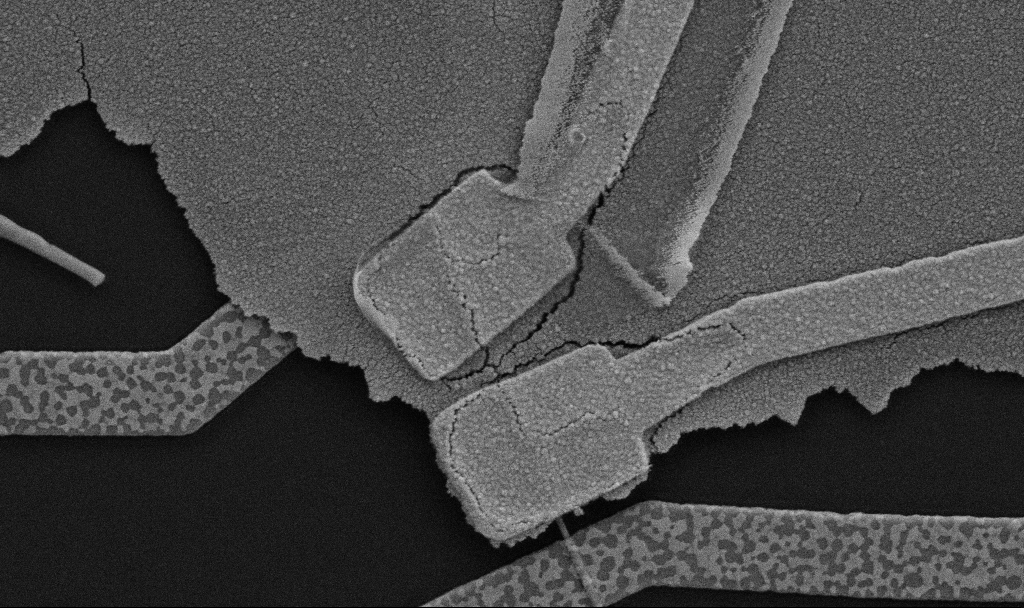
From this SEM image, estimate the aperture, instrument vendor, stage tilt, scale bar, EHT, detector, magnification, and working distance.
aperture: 30 µm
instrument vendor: Zeiss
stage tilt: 0°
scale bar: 1000 nm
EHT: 5 kV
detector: SE2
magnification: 30 K X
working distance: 10.7 mm